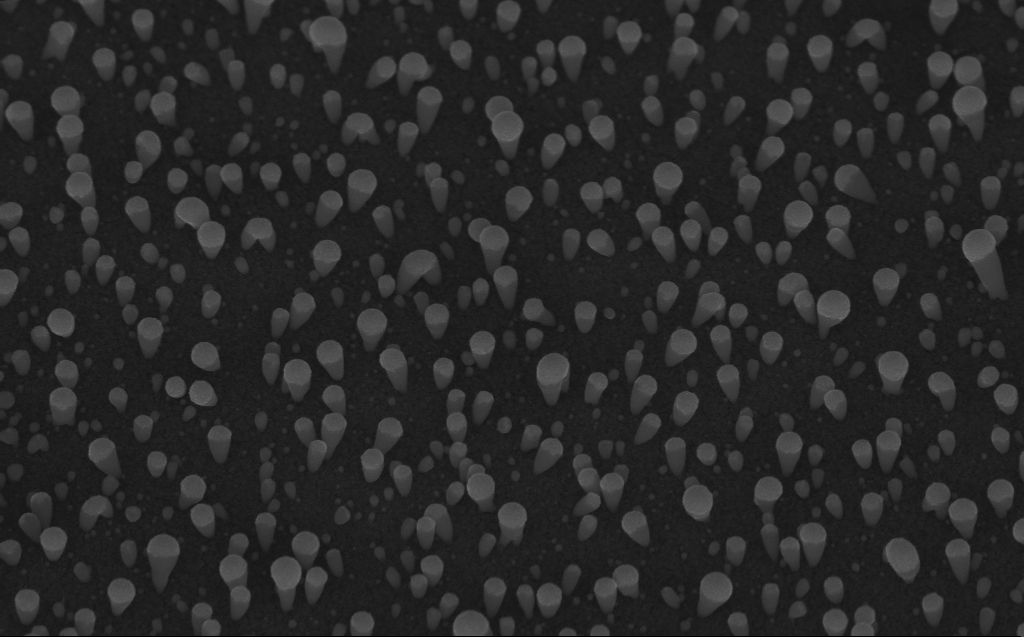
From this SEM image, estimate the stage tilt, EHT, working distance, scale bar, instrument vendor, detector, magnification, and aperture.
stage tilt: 45°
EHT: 10 kV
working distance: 7 mm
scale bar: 1000 nm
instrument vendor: Zeiss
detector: InLens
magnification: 50 K X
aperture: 30 µm